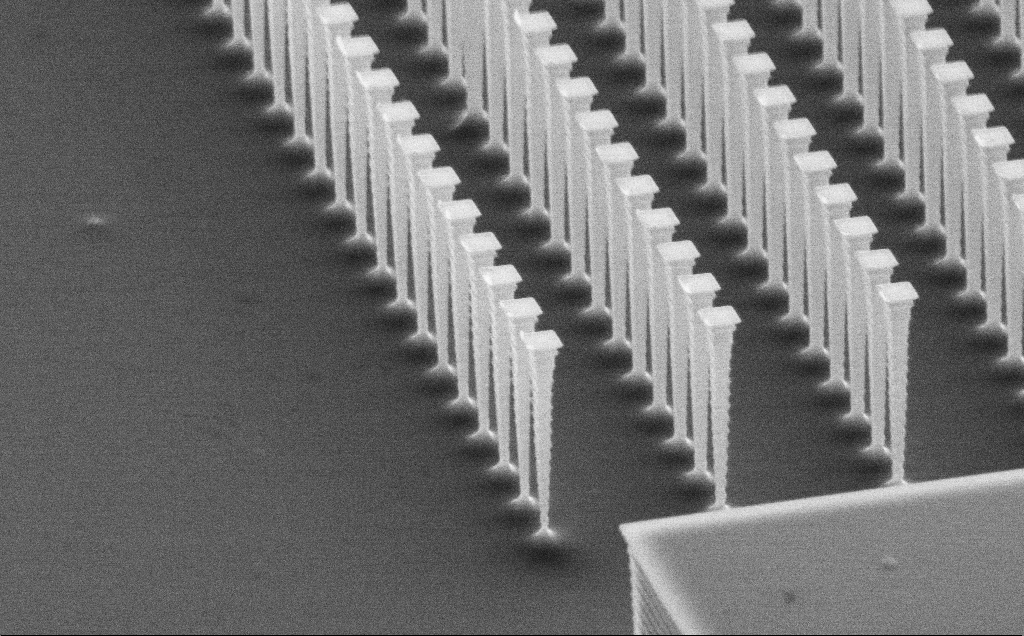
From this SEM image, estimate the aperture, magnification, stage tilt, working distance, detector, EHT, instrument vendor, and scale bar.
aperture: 30 µm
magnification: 13.67 K X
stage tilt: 62°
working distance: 8 mm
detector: SE2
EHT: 10 kV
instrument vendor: Zeiss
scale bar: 1000 nm